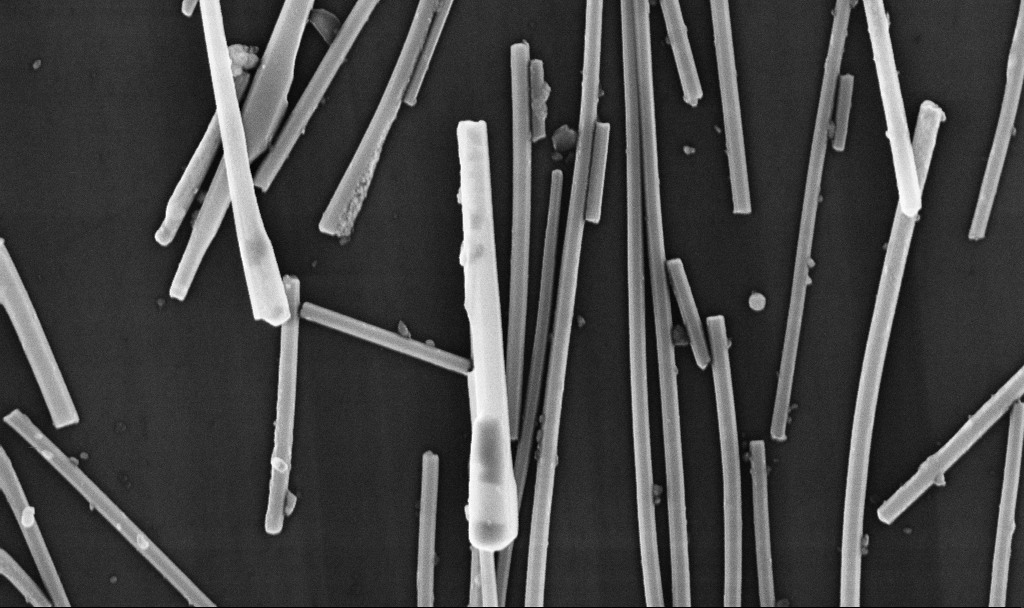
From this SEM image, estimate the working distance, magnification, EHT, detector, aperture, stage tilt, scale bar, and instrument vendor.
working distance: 6.7 mm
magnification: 59.66 K X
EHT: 10 kV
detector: InLens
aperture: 30 µm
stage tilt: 0°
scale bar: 1000 nm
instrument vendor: Zeiss